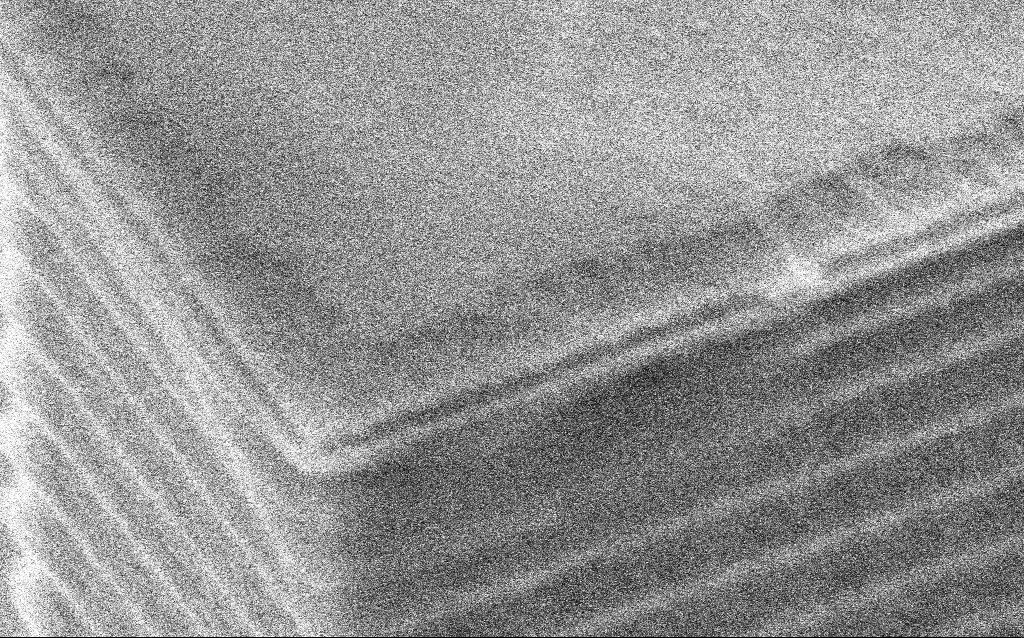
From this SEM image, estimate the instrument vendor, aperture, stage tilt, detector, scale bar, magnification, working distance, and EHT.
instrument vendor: Zeiss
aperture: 30 µm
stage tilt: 45°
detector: SE2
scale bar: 200 nm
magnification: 101.07 K X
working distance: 12 mm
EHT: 5 kV